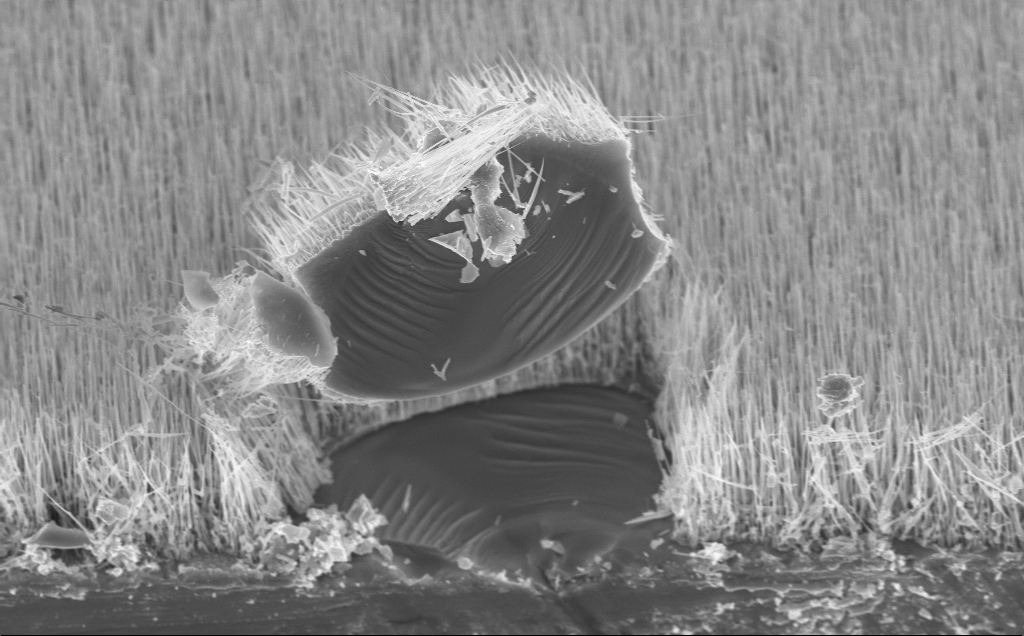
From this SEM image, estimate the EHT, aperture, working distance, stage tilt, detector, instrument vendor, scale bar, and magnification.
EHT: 10 kV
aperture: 30 µm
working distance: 7 mm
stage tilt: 45°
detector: InLens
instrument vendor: Zeiss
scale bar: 2000 nm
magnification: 10.8 K X